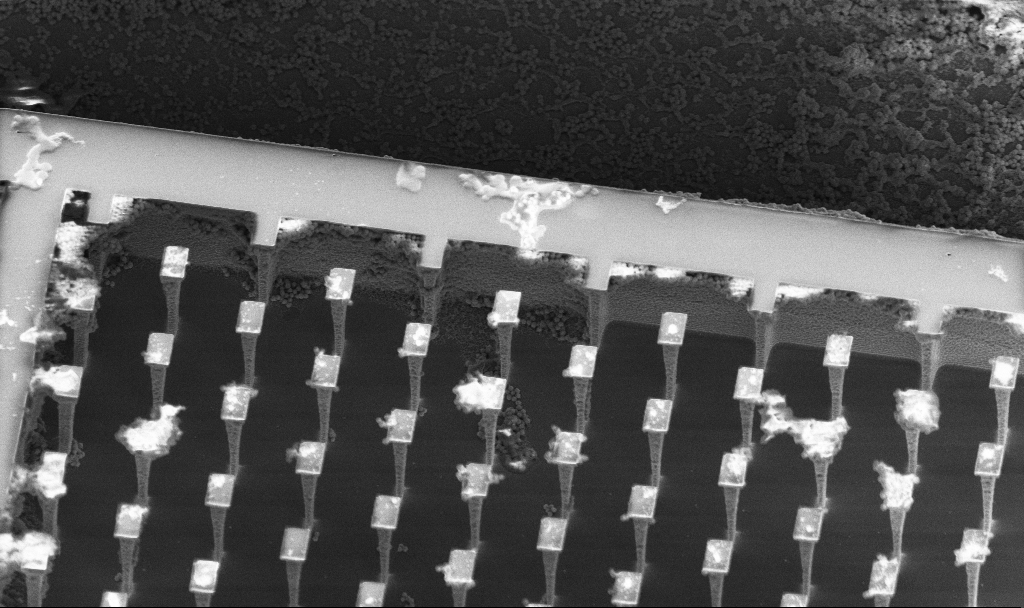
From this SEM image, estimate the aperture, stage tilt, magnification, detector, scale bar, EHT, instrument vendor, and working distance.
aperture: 30 µm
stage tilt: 30°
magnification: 3.07 K X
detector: InLens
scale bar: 10000 nm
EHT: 5 kV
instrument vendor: Zeiss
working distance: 5 mm